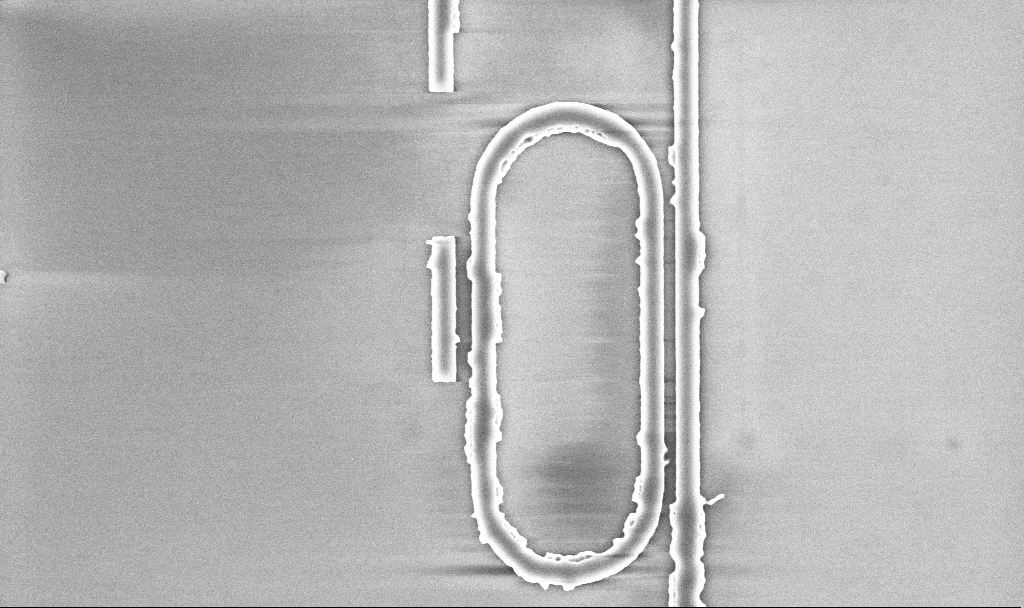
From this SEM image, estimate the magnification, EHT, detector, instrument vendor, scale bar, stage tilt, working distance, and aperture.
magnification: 17.99 K X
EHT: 5 kV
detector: InLens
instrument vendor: Zeiss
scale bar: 2000 nm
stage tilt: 0°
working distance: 5.2 mm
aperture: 30 µm